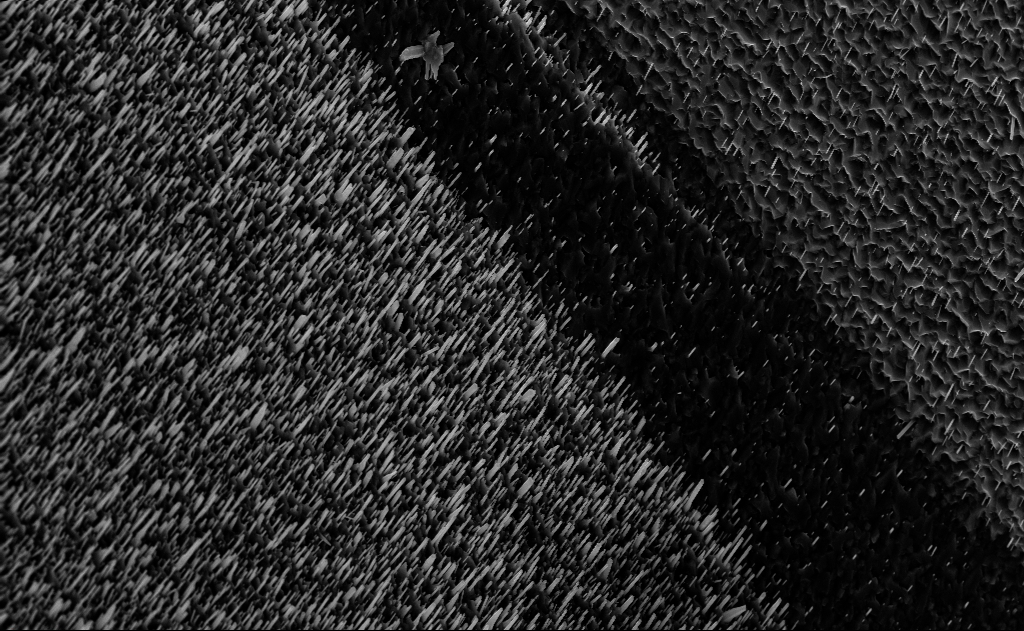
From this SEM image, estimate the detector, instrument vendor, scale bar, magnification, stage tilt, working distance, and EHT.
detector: InLens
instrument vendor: Zeiss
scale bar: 2000 nm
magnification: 10 K X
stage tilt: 0°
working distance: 8 mm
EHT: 10 kV